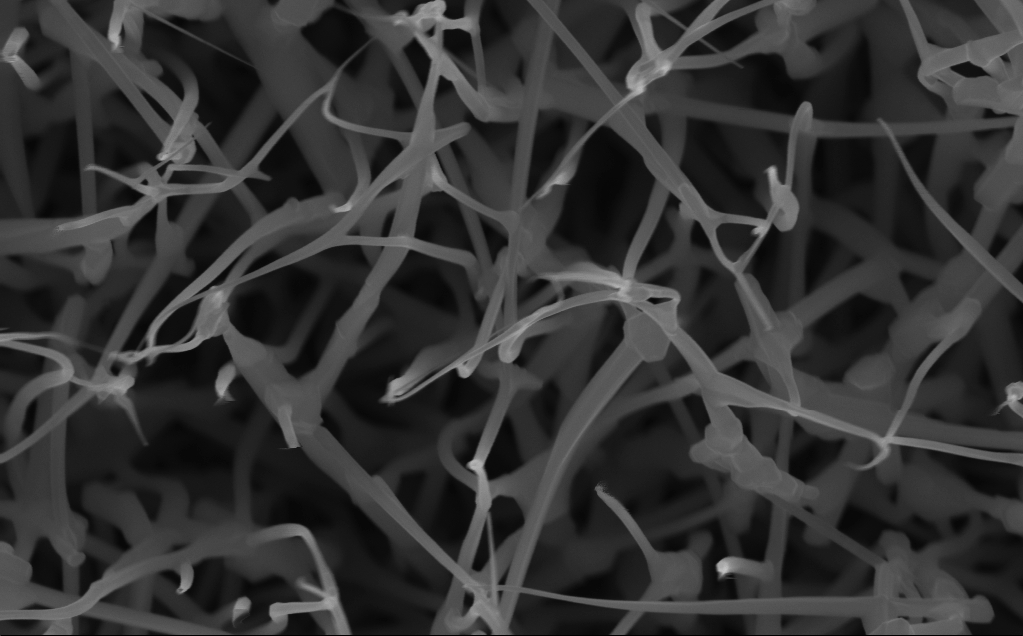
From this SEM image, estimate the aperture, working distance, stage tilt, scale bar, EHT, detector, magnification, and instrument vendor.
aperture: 30 µm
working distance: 5 mm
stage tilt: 0°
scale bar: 200 nm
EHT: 10 kV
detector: InLens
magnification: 80 K X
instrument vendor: Zeiss